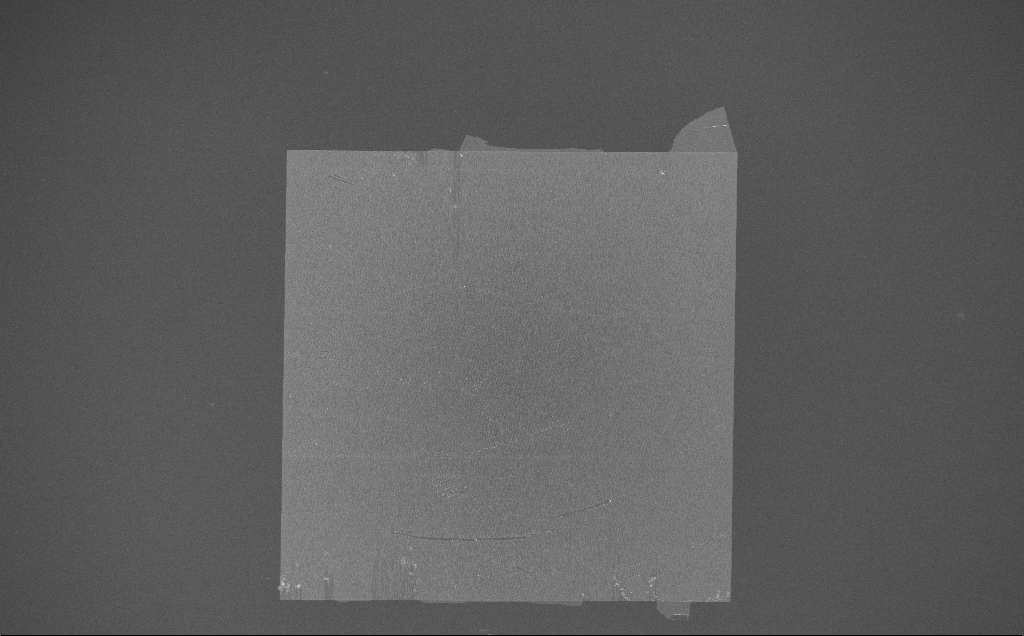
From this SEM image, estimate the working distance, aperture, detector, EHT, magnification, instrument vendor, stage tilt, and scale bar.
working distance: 7 mm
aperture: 30 µm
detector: InLens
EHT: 10 kV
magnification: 0.167 K X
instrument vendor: Zeiss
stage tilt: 0°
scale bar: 100000 nm